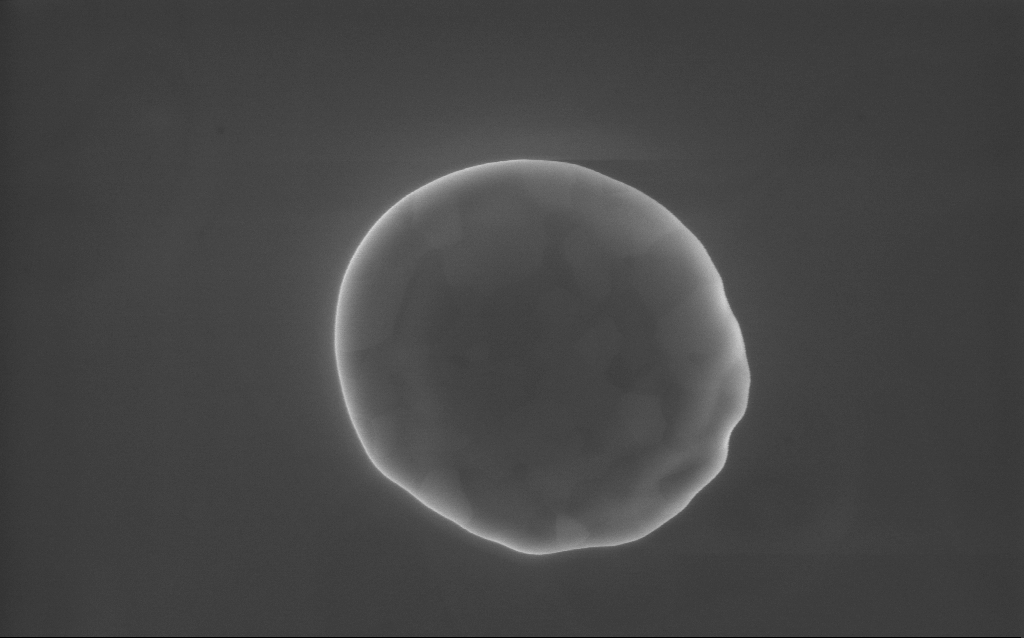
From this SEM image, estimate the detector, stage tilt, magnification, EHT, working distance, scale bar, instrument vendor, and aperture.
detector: InLens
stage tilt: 0°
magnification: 63 K X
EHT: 10 kV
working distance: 3 mm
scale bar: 1000 nm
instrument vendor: Zeiss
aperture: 30 µm